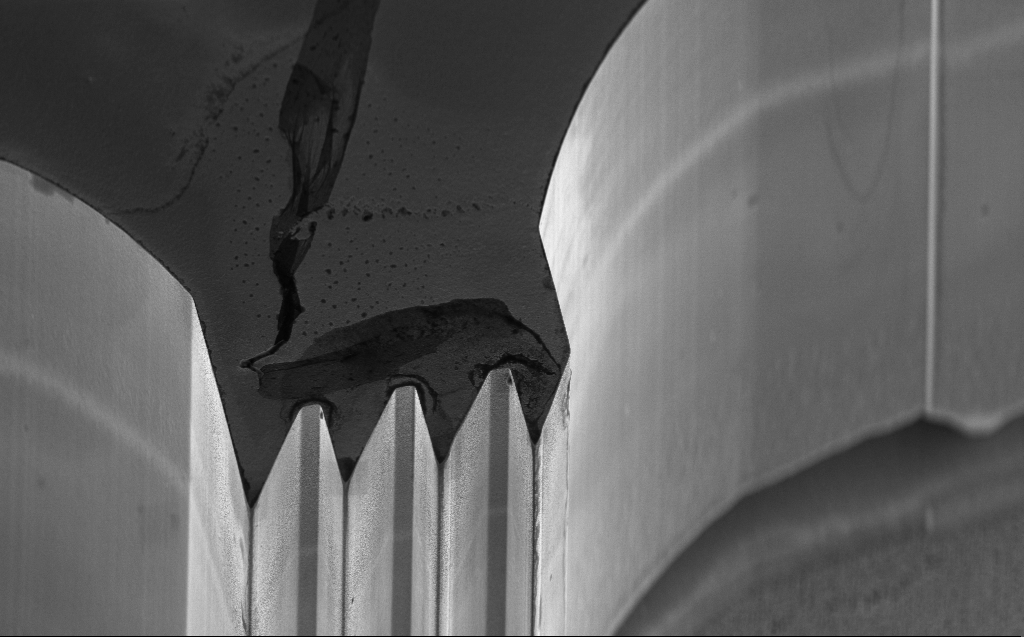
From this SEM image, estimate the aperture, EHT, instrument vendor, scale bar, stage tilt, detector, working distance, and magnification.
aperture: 30 µm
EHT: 5 kV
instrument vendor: Zeiss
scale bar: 10000 nm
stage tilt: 45°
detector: InLens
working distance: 5 mm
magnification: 3.2 K X